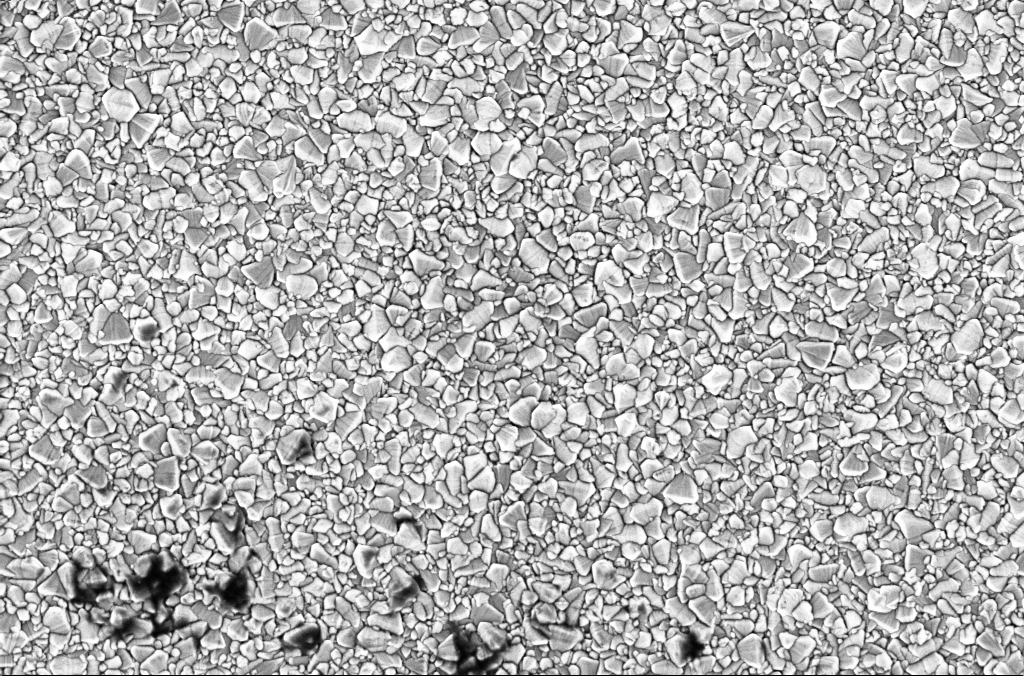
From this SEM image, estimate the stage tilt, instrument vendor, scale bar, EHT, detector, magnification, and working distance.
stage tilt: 0°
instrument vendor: Zeiss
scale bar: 2000 nm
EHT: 2 kV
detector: InLens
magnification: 30 K X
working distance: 1.9 mm